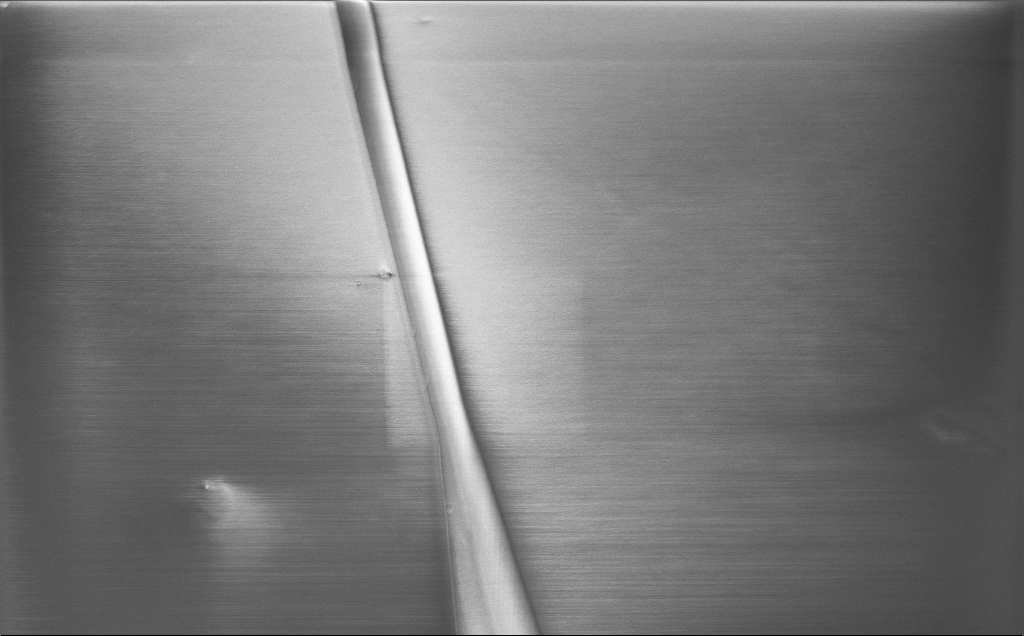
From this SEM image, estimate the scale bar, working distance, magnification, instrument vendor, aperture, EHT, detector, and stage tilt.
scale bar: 10000 nm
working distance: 6 mm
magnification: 3.72 K X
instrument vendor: Zeiss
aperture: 30 µm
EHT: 1.2 kV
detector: InLens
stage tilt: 40°